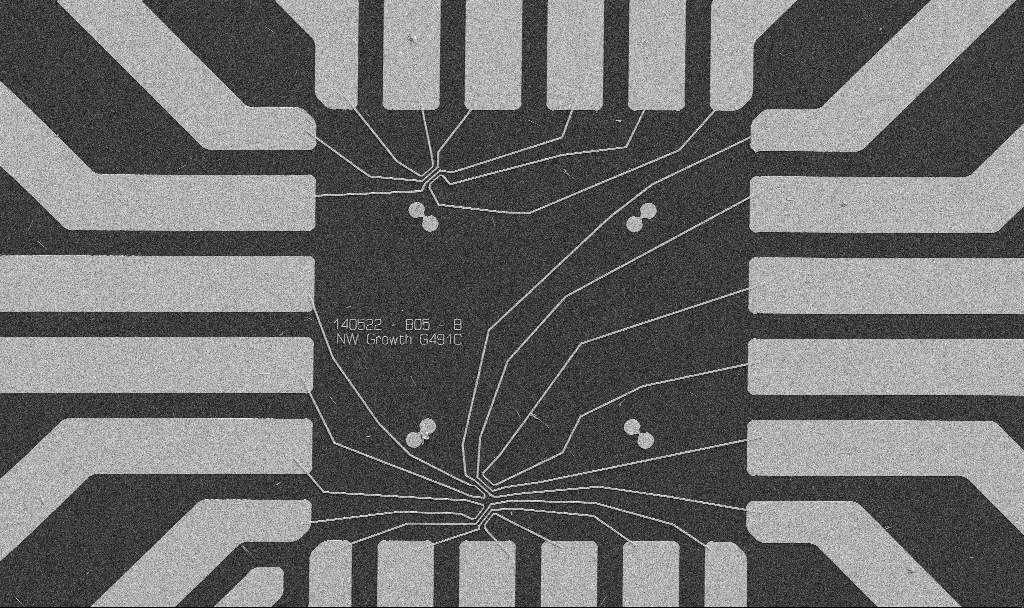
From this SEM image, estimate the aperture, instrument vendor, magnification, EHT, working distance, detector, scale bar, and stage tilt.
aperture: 30 µm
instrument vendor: Zeiss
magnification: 1 K X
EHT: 5 kV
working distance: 10.7 mm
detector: SE2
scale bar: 20000 nm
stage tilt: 0°